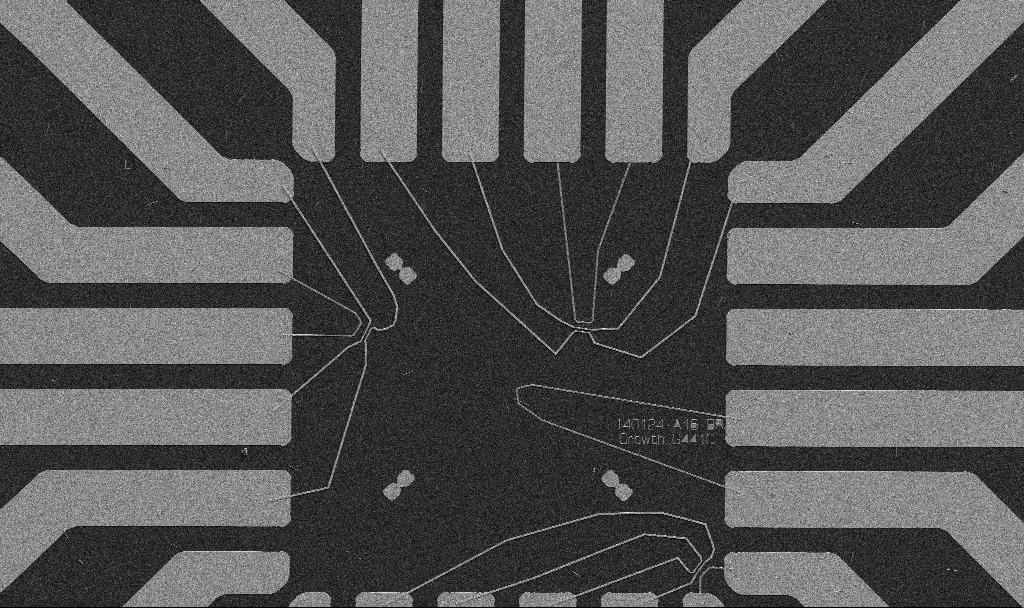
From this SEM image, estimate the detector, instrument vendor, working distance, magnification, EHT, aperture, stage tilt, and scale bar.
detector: SE2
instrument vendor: Zeiss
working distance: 10.7 mm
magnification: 1 K X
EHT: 5 kV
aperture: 30 µm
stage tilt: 0°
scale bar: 20000 nm